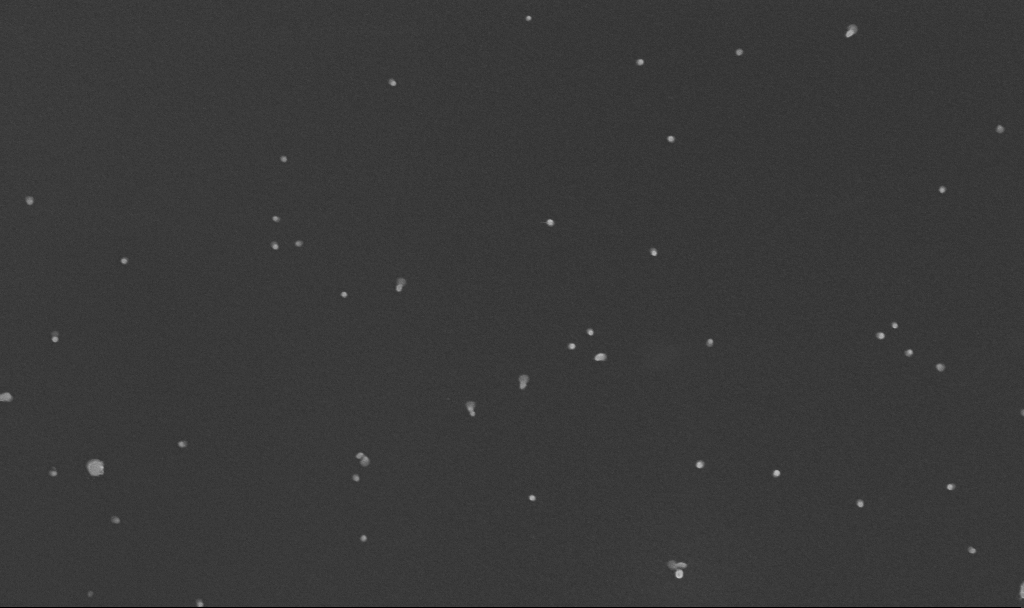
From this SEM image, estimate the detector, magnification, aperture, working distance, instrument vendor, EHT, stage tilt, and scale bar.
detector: InLens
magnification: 100 K X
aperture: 30 µm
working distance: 3.3 mm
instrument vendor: Zeiss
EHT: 10 kV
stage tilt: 0°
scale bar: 200 nm